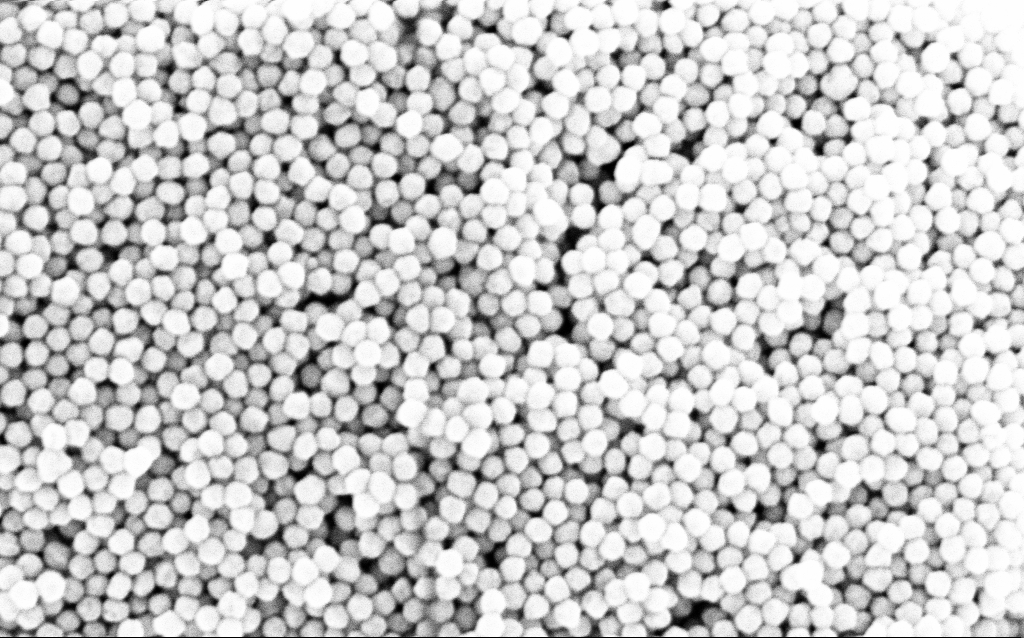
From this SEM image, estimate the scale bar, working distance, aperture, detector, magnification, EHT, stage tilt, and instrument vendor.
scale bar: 200 nm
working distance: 3.2 mm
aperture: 30 µm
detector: InLens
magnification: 157.97 K X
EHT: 8 kV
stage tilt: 0°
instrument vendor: Zeiss